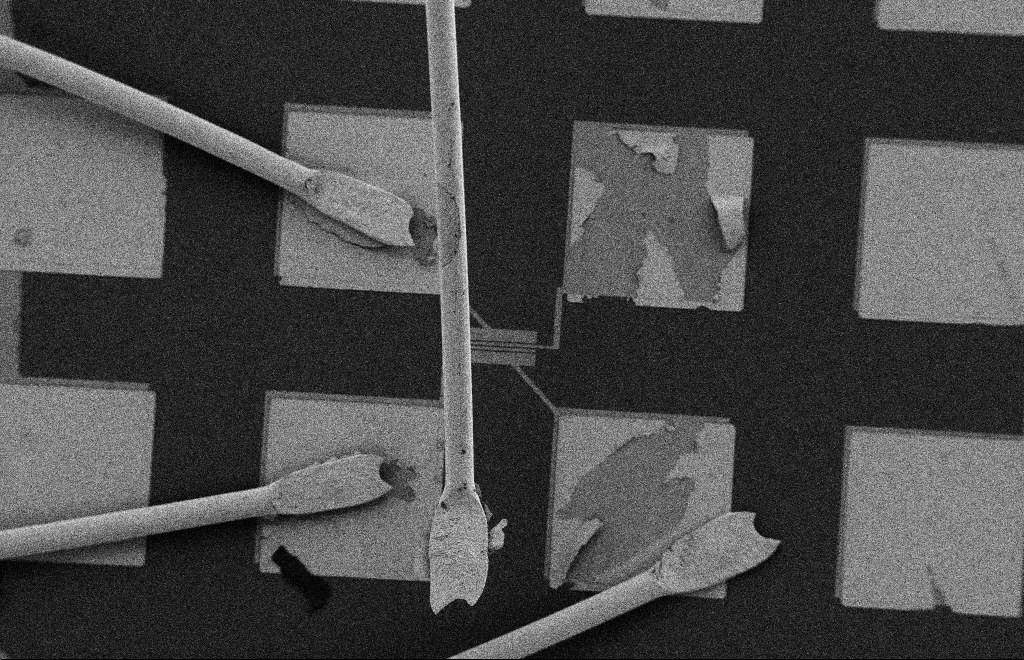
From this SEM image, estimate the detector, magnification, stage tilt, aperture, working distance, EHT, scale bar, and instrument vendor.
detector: SE2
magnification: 0.421 K X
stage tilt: -0.3°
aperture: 20 µm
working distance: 10 mm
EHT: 2 kV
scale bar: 100000 nm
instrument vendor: Zeiss